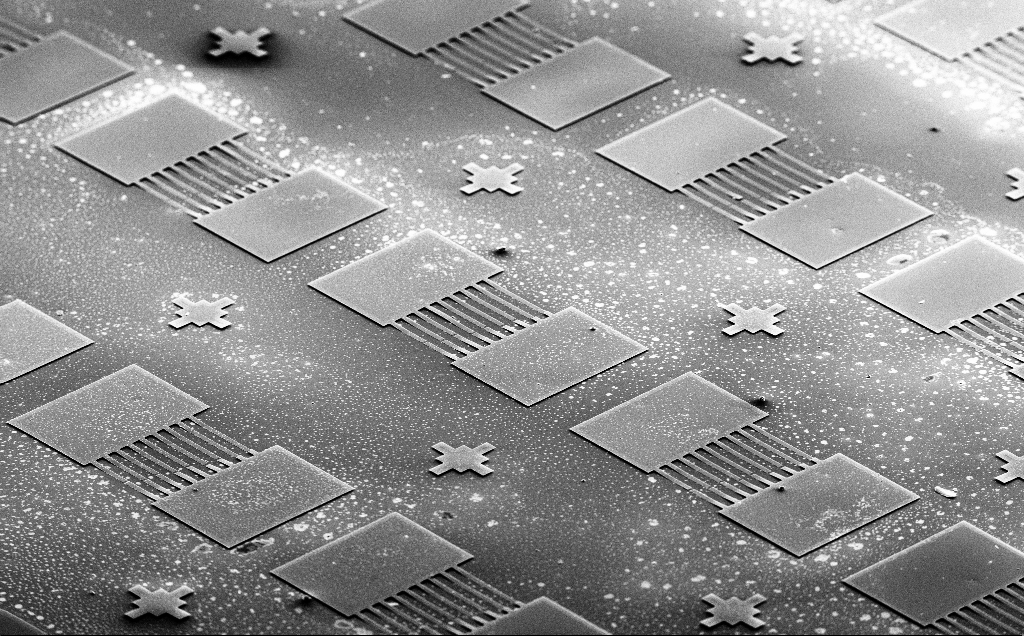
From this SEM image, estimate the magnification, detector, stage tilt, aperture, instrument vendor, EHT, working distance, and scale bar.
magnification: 0.147 K X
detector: SE2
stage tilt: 59.7°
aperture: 30 µm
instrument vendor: Zeiss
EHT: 10 kV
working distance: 14 mm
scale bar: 100000 nm